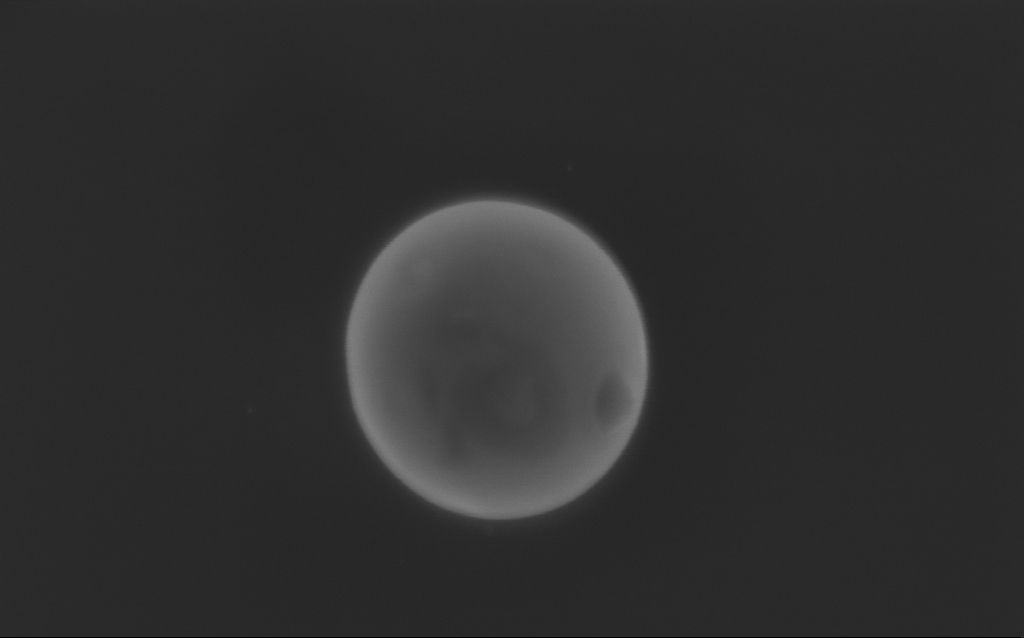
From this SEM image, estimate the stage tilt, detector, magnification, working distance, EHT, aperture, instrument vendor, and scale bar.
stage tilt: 0°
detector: InLens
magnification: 121.03 K X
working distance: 4 mm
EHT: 3 kV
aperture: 30 µm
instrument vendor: Zeiss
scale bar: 200 nm